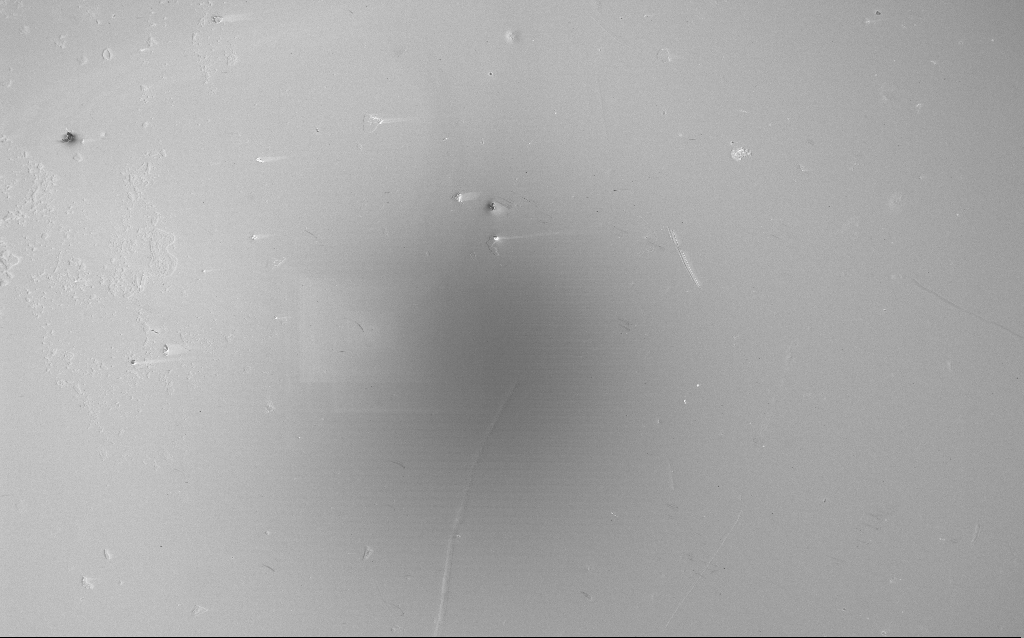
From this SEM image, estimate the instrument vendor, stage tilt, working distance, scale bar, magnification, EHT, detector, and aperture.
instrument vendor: Zeiss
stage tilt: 0°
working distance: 4 mm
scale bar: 100000 nm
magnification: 0.172 K X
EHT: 5 kV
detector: InLens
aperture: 30 µm